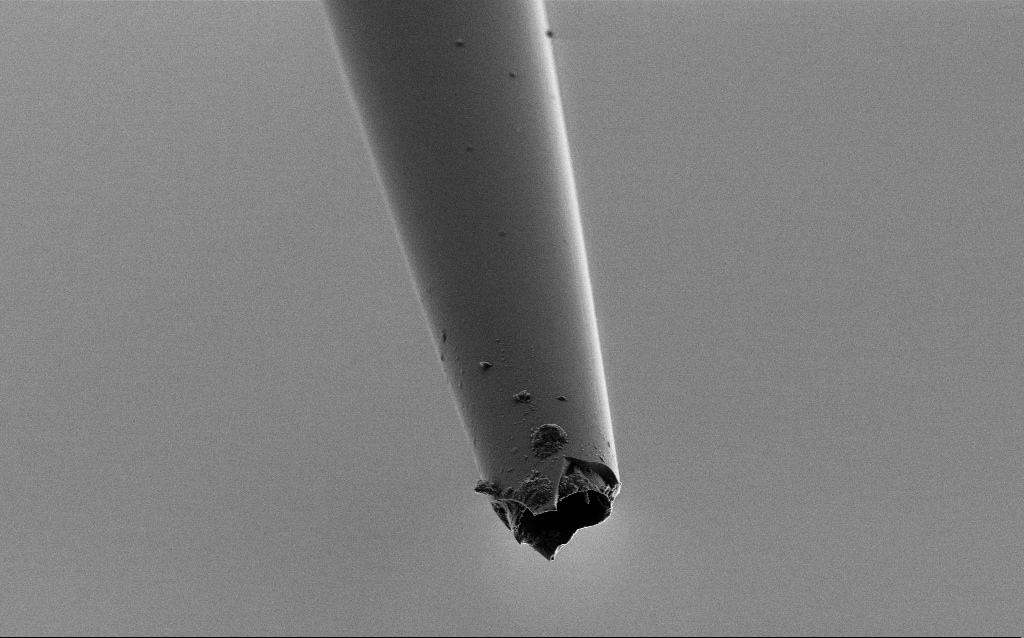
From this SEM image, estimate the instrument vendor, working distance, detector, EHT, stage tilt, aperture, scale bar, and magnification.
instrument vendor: Zeiss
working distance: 6 mm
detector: SE2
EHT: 2 kV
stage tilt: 45°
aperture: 30 µm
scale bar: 10000 nm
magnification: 5 K X